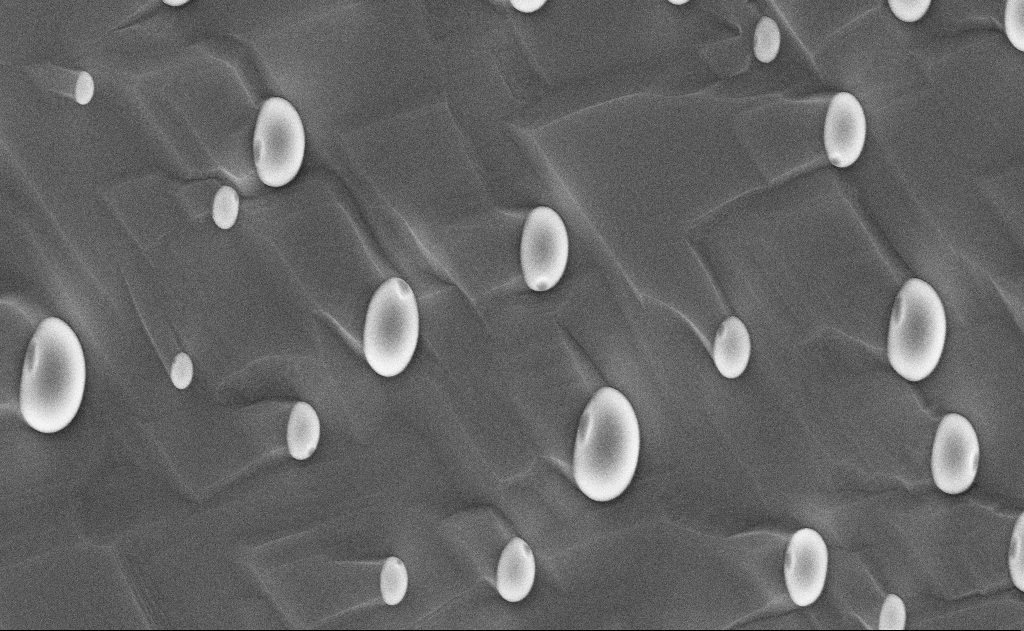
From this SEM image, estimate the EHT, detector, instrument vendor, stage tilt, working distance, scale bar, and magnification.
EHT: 10 kV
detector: InLens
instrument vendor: Zeiss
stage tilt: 0°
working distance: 15 mm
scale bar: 2000 nm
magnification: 20 K X